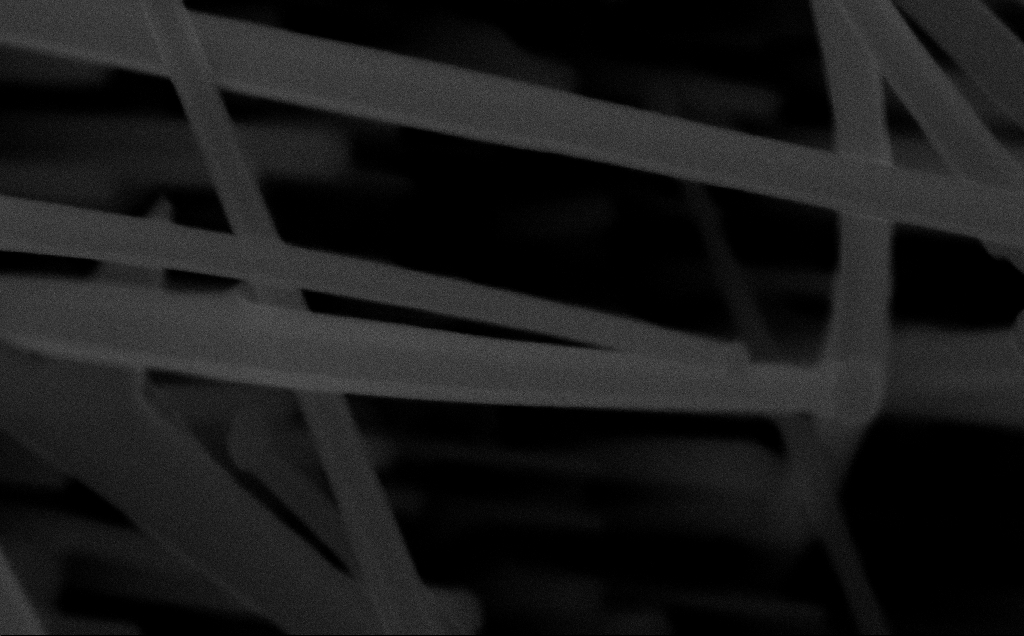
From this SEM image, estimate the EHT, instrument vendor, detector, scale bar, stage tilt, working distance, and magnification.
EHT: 10 kV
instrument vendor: Zeiss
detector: InLens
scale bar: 200 nm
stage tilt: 0°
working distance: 6 mm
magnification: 151.53 K X